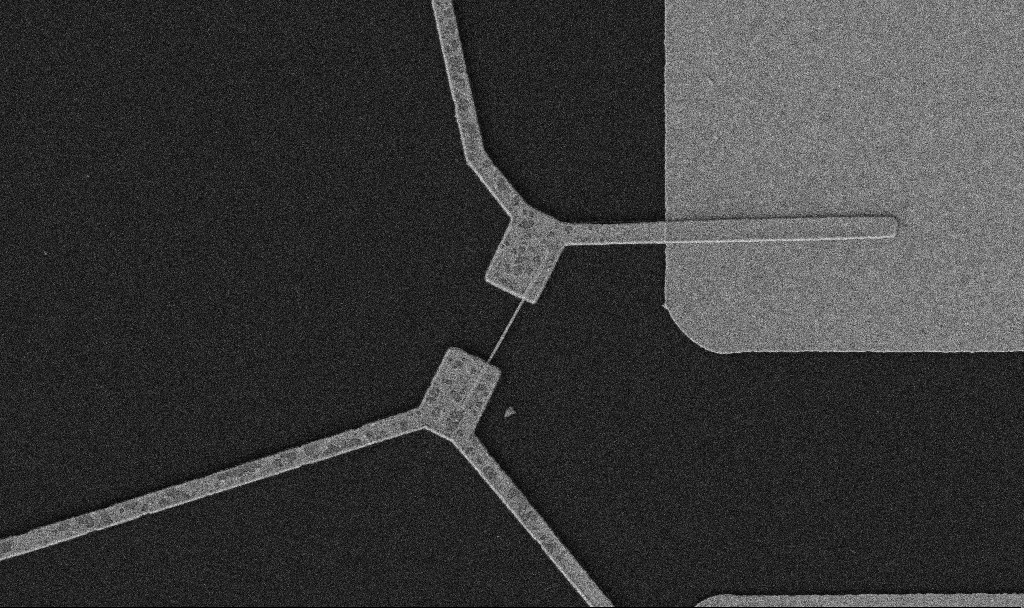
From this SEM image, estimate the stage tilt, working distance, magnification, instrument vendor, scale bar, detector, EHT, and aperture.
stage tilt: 0°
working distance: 10.7 mm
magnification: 10 K X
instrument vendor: Zeiss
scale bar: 2000 nm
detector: SE2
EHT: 5 kV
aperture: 30 µm